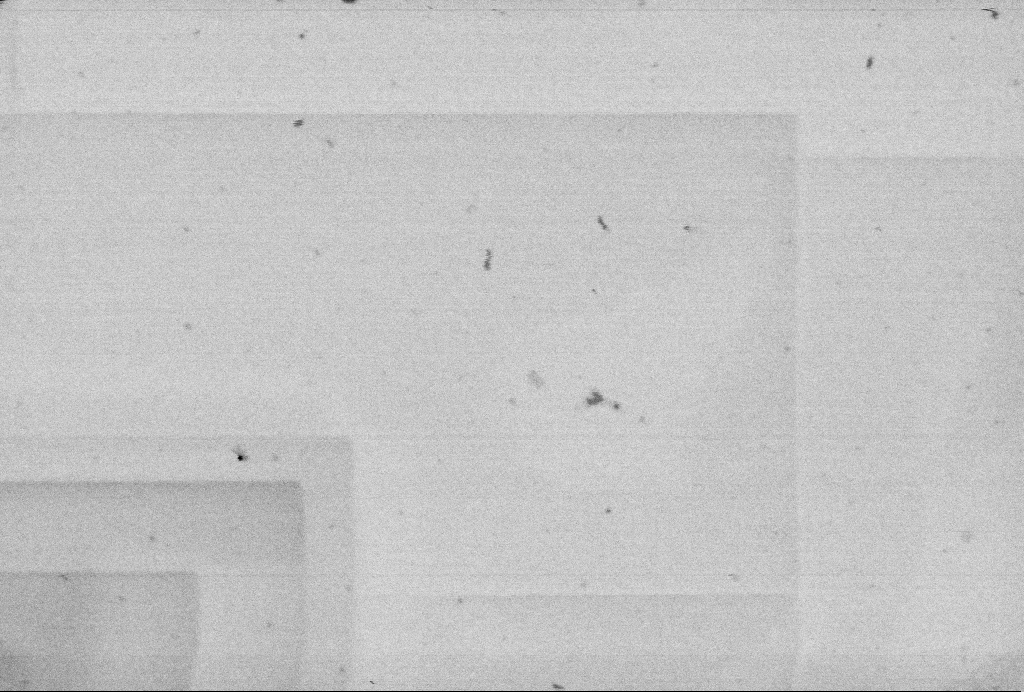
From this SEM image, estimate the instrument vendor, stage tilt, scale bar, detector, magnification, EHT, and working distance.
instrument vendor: Zeiss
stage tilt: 0°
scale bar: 200 nm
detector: SE2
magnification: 67.74 K X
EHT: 1 kV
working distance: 5 mm